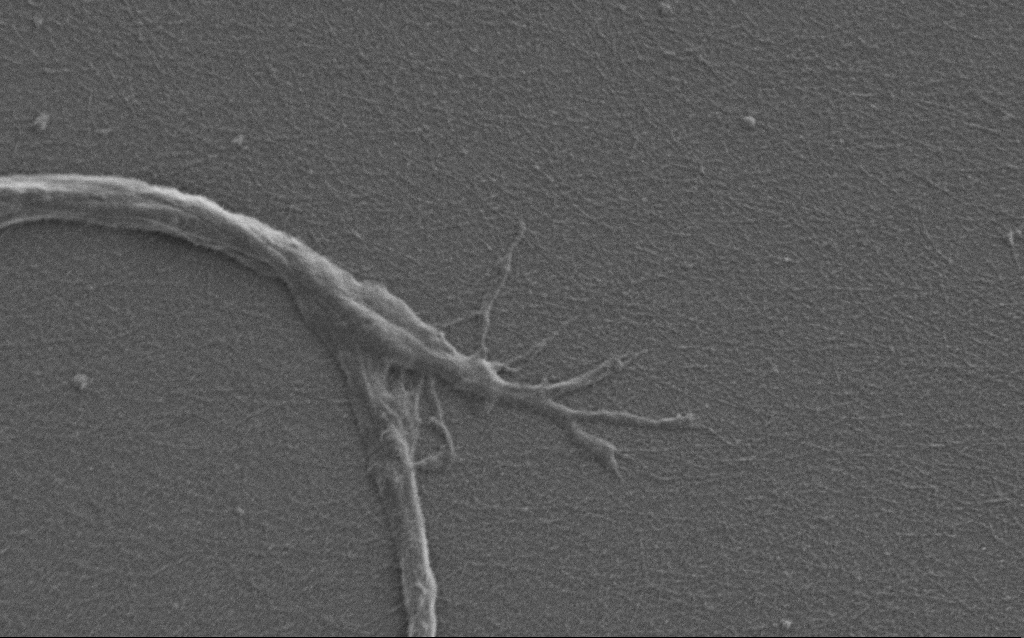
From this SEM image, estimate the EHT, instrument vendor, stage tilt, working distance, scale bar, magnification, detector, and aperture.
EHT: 0.9 kV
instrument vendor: Zeiss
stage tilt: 0°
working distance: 7 mm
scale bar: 2000 nm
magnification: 10 K X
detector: SE2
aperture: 30 µm